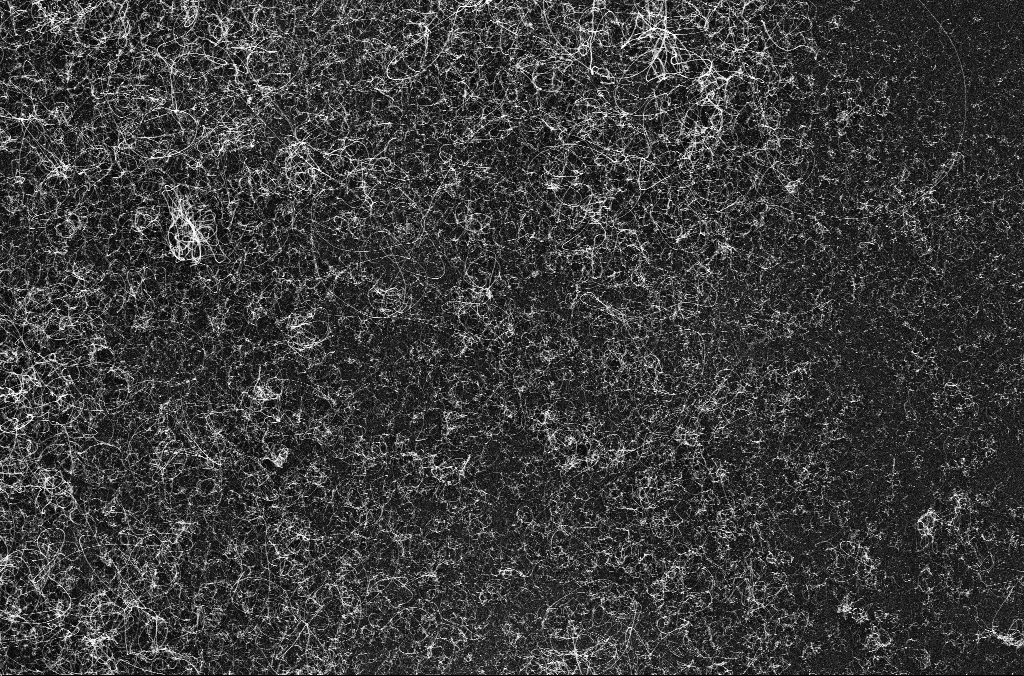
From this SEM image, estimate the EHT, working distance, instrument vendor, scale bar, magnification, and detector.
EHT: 10 kV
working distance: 3.3 mm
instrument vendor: Zeiss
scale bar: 2000 nm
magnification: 10 K X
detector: InLens